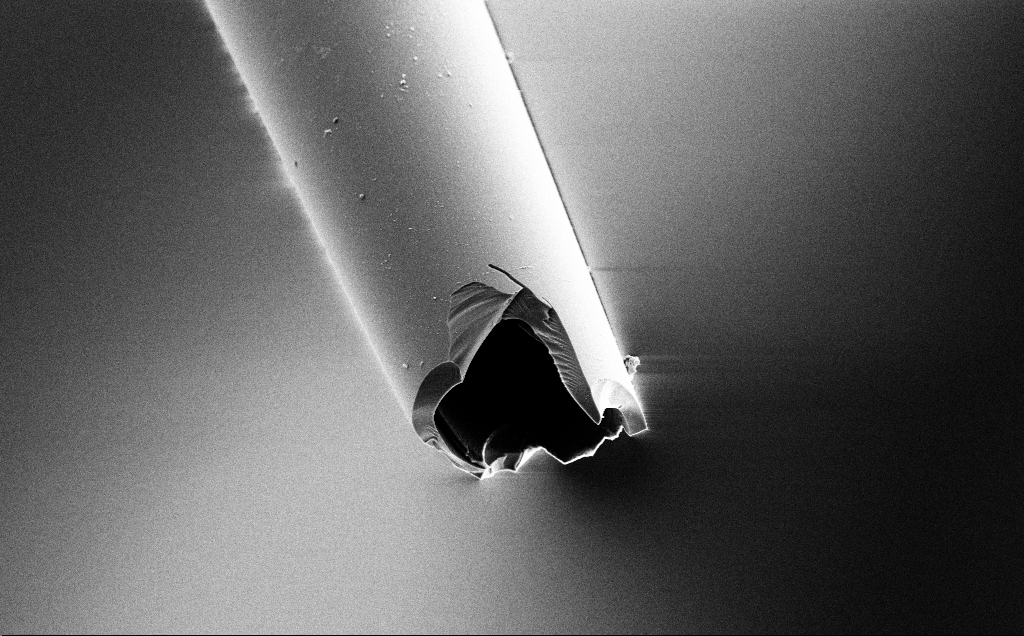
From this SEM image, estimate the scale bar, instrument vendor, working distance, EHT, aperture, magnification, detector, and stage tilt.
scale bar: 10000 nm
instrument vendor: Zeiss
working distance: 7.6 mm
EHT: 3 kV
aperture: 30 µm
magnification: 5 K X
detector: SE2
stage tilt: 45°